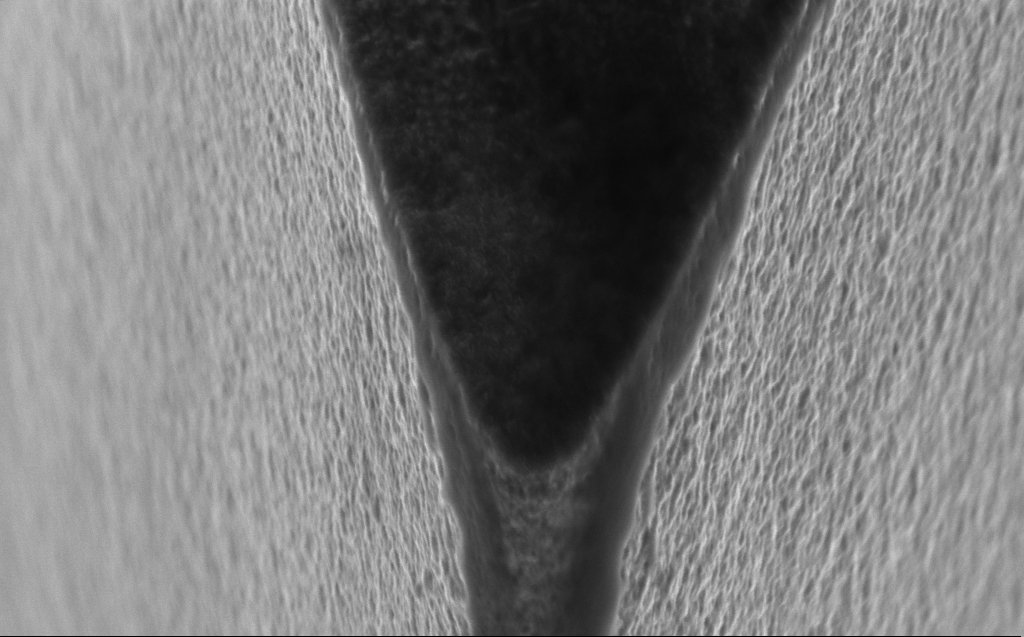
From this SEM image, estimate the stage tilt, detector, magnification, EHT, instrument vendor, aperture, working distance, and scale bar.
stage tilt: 45°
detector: InLens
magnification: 73.21 K X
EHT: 5 kV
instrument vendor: Zeiss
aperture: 30 µm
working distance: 5 mm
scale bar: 200 nm